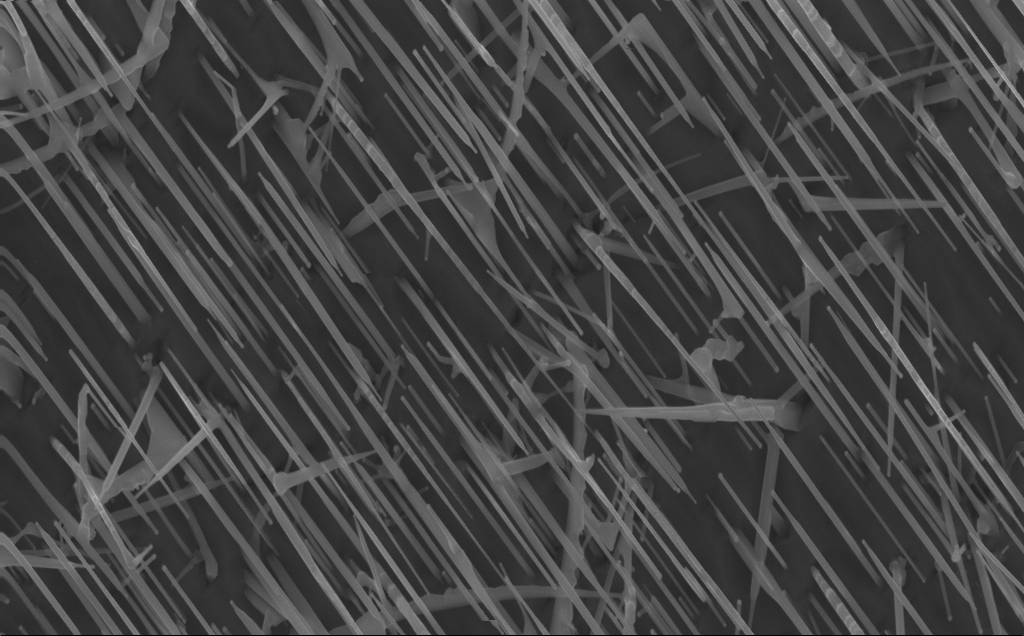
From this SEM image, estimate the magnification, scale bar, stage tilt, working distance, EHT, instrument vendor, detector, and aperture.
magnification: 40 K X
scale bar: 1000 nm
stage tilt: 0°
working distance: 4 mm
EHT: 10 kV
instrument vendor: Zeiss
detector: InLens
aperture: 30 µm